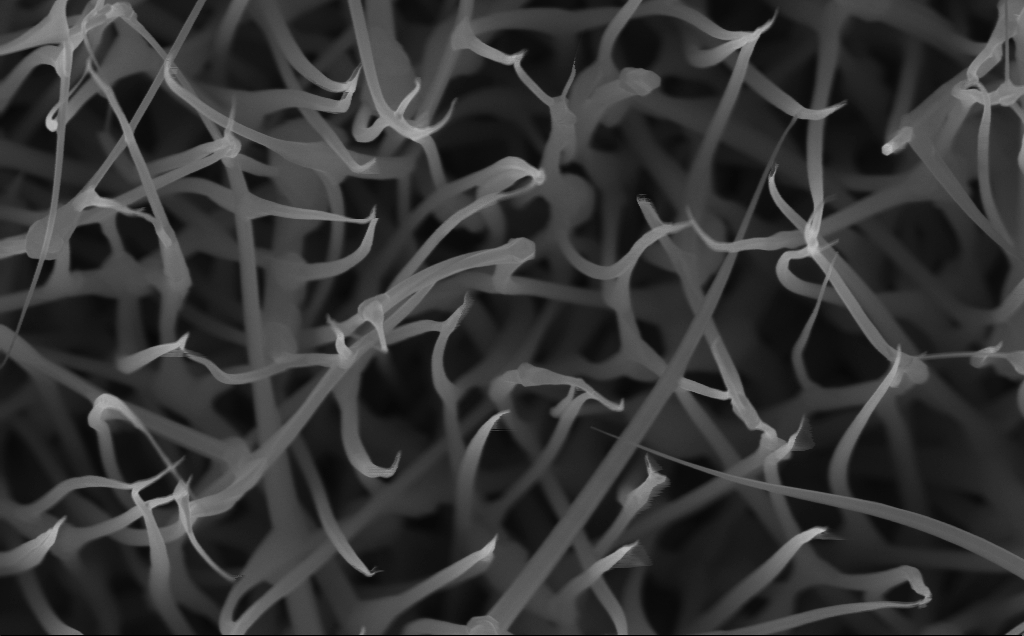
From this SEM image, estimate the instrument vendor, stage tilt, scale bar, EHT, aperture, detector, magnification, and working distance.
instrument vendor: Zeiss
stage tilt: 0°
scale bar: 200 nm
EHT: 10 kV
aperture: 30 µm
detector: InLens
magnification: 80 K X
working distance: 4 mm